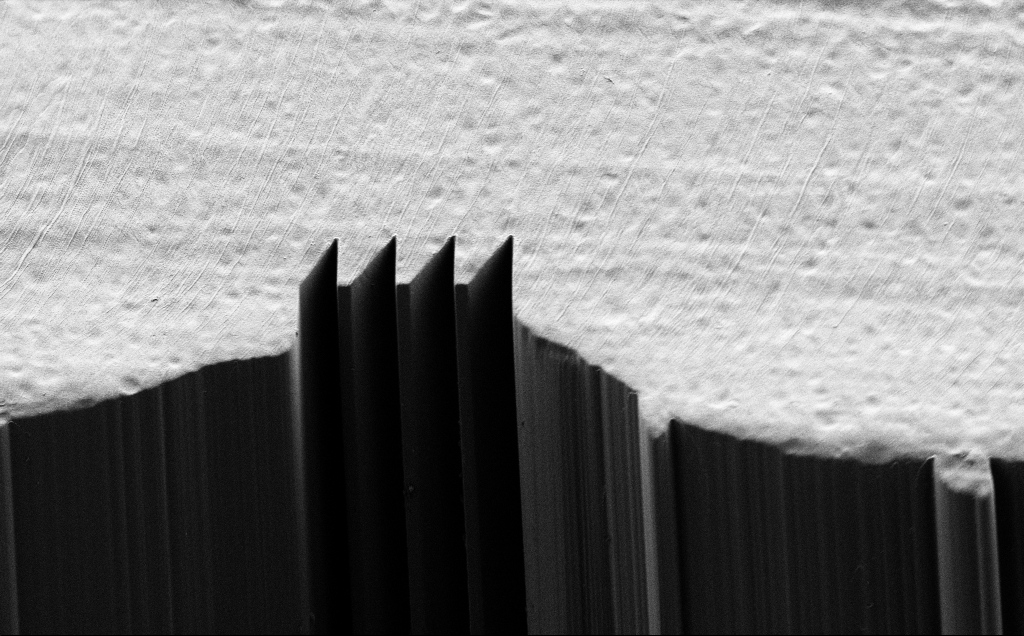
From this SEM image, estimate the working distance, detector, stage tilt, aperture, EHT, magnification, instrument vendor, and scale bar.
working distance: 8 mm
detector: SE2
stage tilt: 45°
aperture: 30 µm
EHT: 1.2 kV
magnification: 1.85 K X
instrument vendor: Zeiss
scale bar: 20000 nm